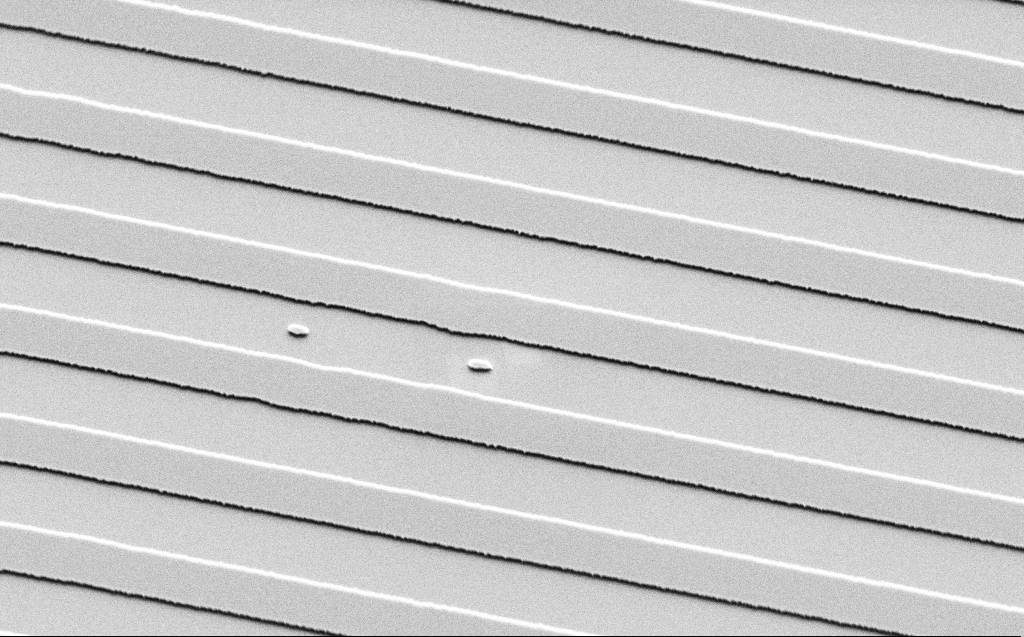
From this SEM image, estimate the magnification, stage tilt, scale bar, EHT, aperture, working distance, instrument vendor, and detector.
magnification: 9.97 K X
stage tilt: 45°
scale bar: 2000 nm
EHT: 3 kV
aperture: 30 µm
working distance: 9 mm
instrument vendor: Zeiss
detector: SE2